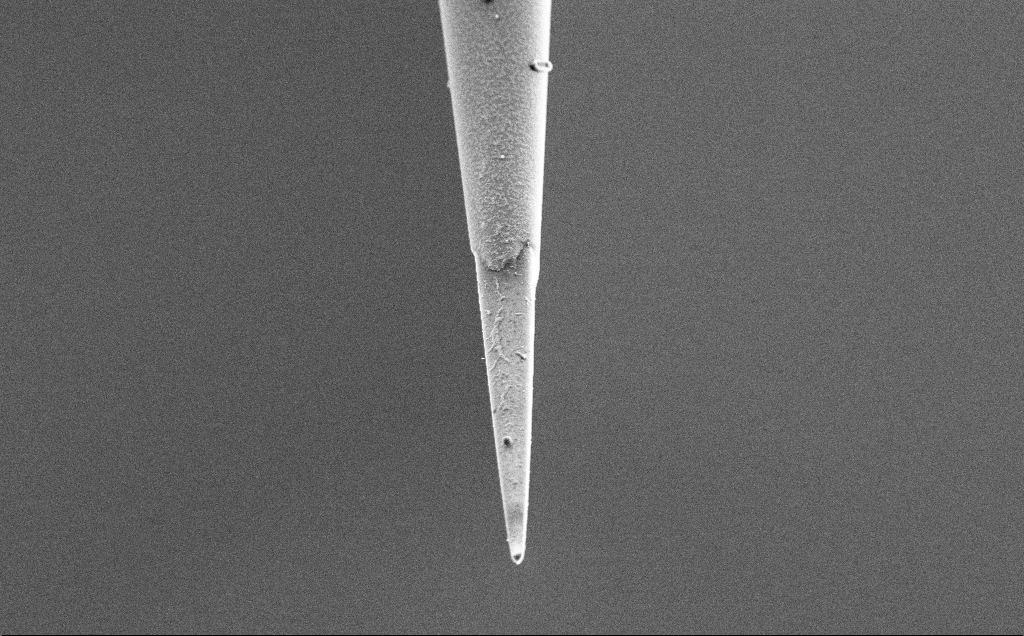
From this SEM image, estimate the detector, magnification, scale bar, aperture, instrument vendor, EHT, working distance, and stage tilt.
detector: SE2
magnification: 7.5 K X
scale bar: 2000 nm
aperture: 30 µm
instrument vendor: Zeiss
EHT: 3 kV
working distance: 7.5 mm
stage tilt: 45°